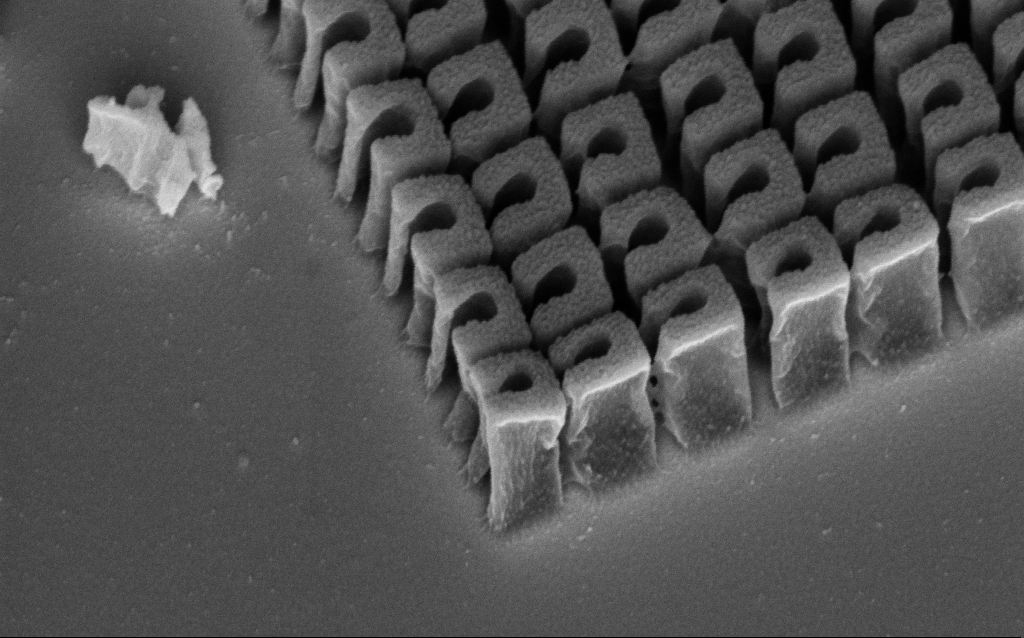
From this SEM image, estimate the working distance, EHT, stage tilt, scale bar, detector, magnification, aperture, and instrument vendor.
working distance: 7.3 mm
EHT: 3 kV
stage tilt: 45°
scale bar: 200 nm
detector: InLens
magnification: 83.3 K X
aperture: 30 µm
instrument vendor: Zeiss